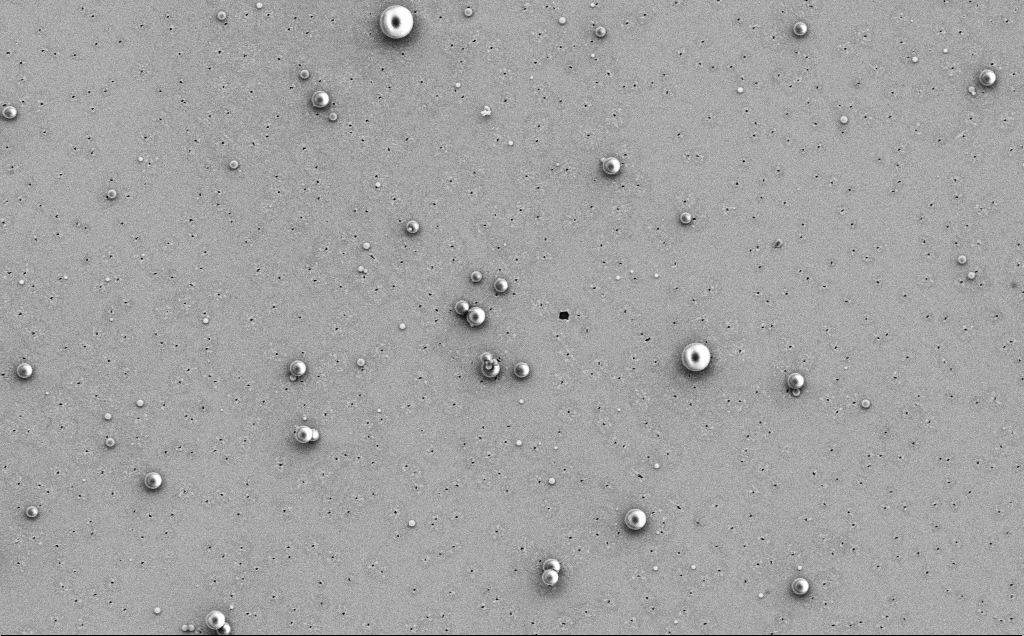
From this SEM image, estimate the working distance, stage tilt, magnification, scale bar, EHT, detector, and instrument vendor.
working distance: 12 mm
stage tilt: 0°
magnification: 4.2 K X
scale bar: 10000 nm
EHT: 5 kV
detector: SE2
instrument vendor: Zeiss